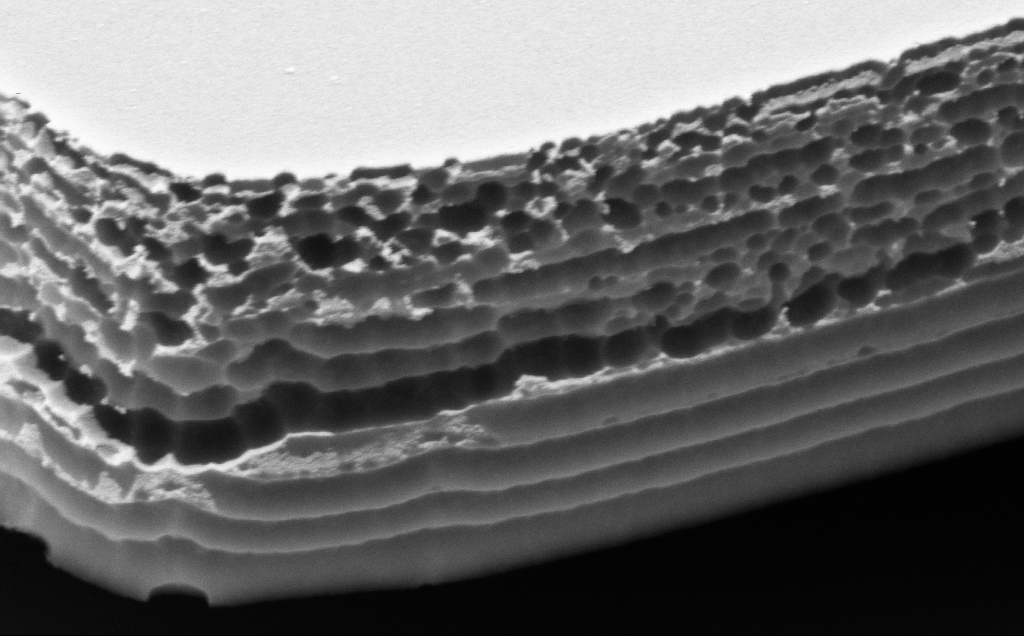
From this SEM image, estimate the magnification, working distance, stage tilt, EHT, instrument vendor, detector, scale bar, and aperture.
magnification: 79.85 K X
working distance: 10 mm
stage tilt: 50°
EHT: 5 kV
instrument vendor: Zeiss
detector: SE2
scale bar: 200 nm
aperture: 30 µm